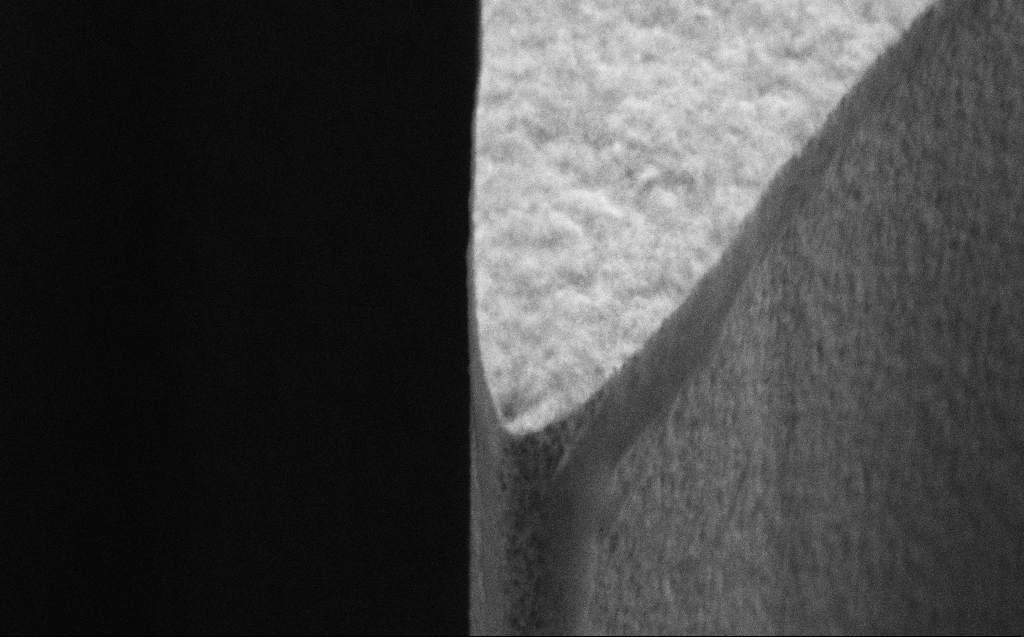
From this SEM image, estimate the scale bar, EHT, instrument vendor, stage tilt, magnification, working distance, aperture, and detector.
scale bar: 1000 nm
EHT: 5 kV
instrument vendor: Zeiss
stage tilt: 45°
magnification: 51.05 K X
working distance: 5 mm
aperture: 30 µm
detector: SE2